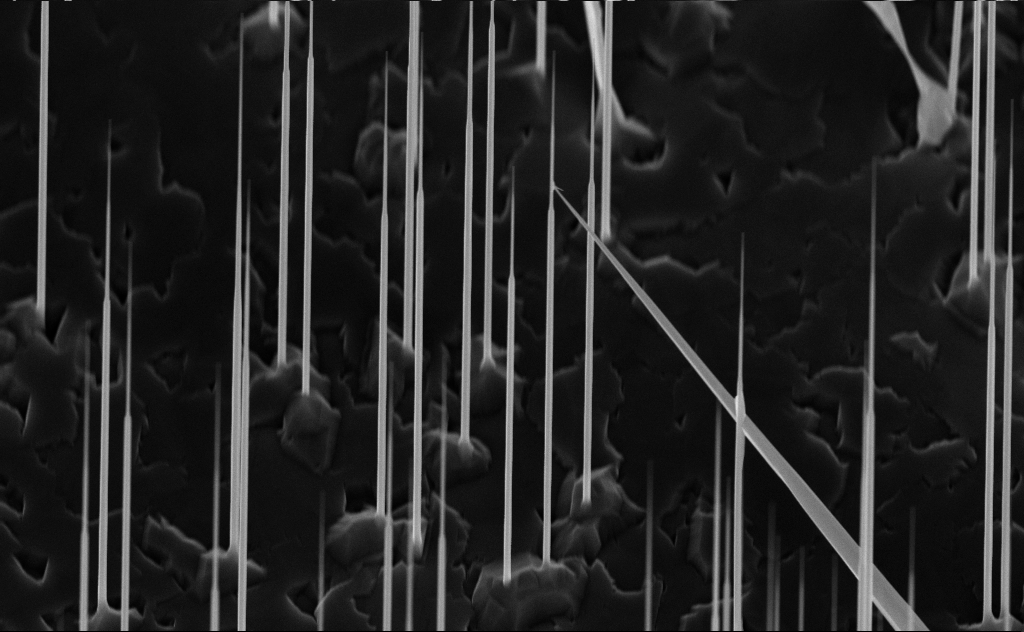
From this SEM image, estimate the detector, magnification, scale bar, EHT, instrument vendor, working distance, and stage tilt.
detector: InLens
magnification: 20 K X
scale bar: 2000 nm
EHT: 10 kV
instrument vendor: Zeiss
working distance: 6 mm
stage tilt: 45°